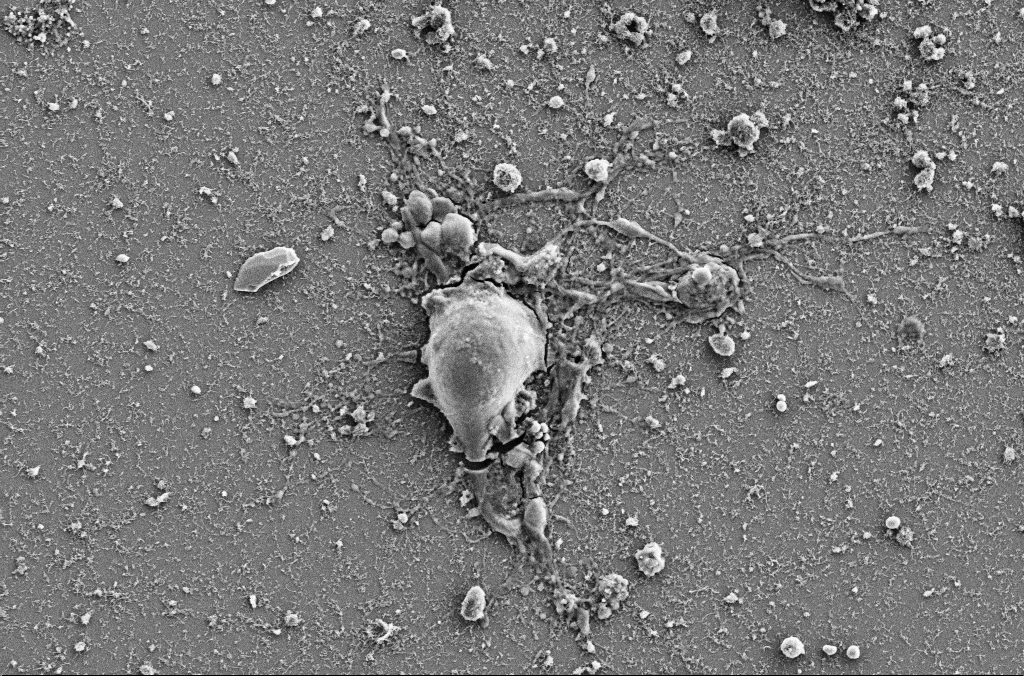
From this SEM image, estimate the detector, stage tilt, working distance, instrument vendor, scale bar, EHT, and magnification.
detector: SE2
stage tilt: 0°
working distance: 4 mm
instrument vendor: Zeiss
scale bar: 10000 nm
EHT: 5 kV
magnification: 4 K X